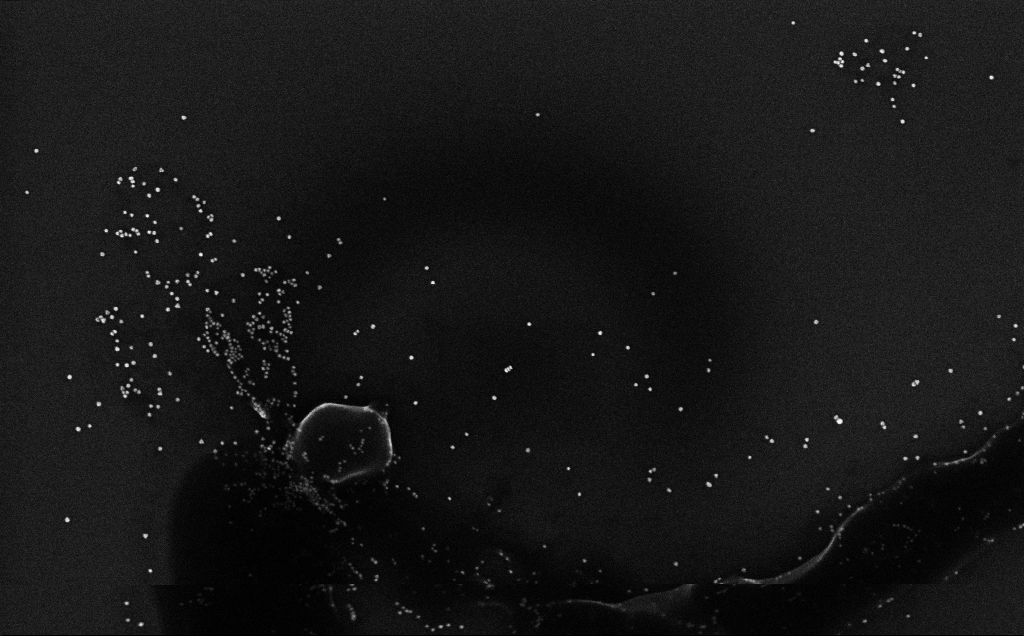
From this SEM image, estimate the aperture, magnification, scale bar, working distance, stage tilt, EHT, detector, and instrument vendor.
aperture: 30 µm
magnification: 100 K X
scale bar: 200 nm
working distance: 3.2 mm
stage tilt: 0°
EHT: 10 kV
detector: InLens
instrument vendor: Zeiss